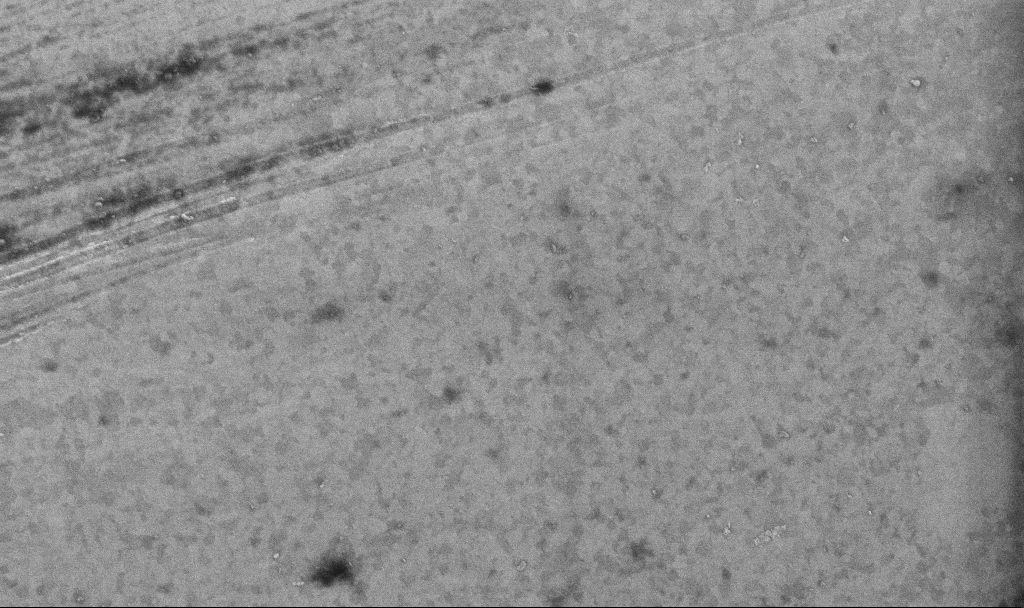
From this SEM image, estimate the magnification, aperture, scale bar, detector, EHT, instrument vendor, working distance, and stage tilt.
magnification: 50.94 K X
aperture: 30 µm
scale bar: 1000 nm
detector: InLens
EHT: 10 kV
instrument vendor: Zeiss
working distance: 3.4 mm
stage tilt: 0°